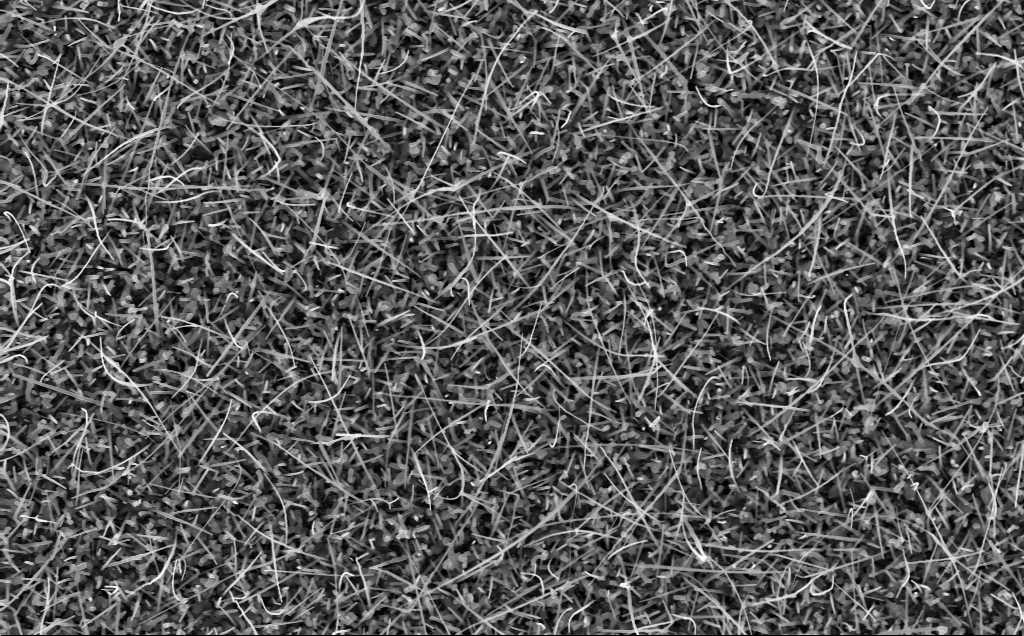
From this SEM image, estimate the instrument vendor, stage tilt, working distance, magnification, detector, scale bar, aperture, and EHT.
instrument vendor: Zeiss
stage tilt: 0°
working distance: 6 mm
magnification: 20 K X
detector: InLens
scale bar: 1000 nm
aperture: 30 µm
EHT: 10 kV